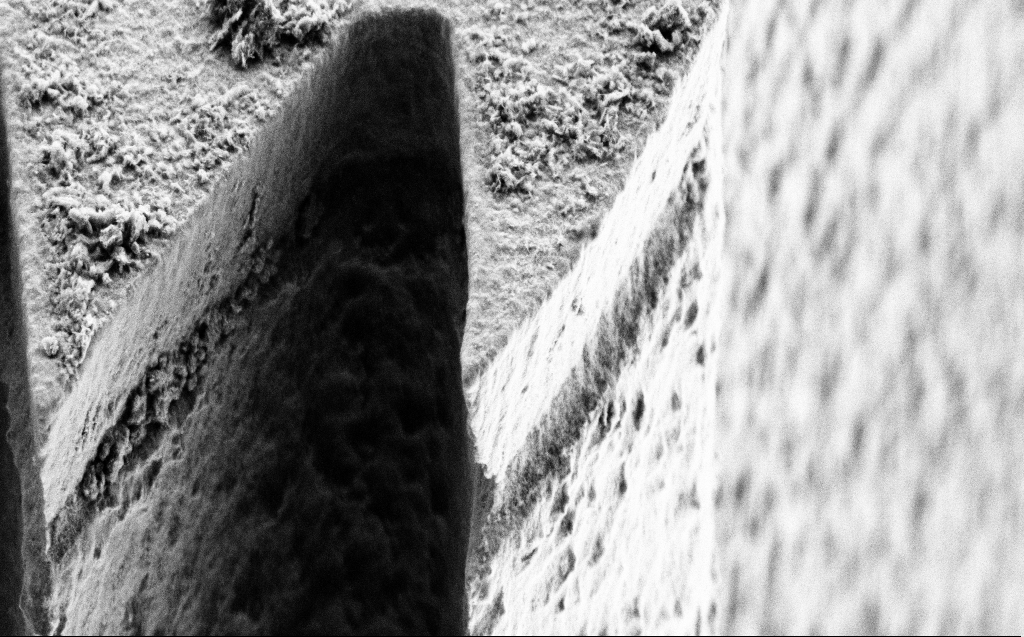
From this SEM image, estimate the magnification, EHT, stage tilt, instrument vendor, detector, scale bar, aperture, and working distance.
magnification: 14.54 K X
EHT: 3 kV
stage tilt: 45°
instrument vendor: Zeiss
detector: SE2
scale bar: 2000 nm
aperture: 30 µm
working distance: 6 mm